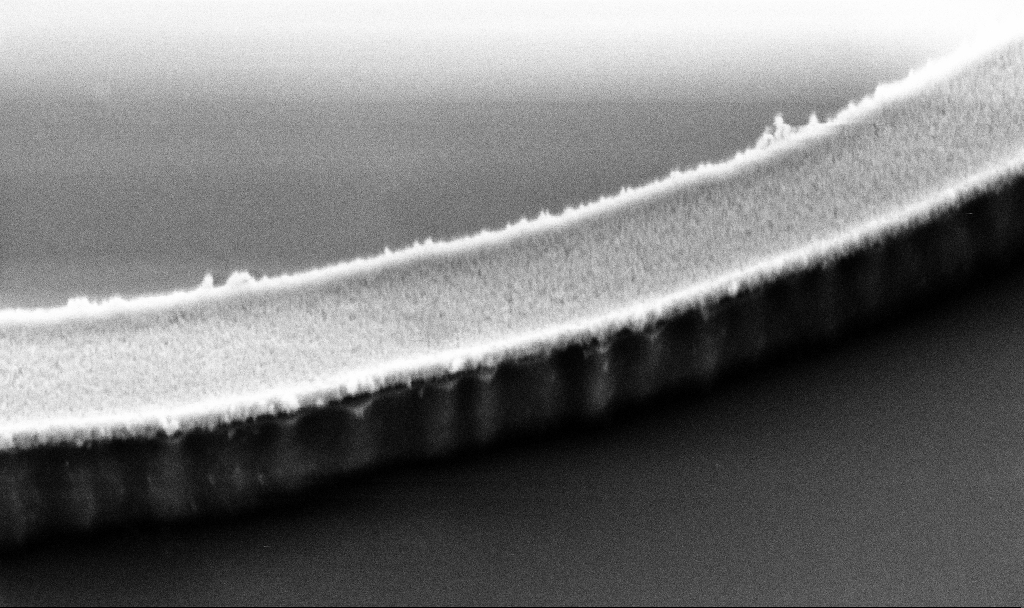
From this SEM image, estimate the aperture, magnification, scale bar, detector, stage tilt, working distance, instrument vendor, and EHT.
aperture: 30 µm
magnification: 98.61 K X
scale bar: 200 nm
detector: SE2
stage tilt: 45°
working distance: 7.2 mm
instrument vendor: Zeiss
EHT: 3 kV